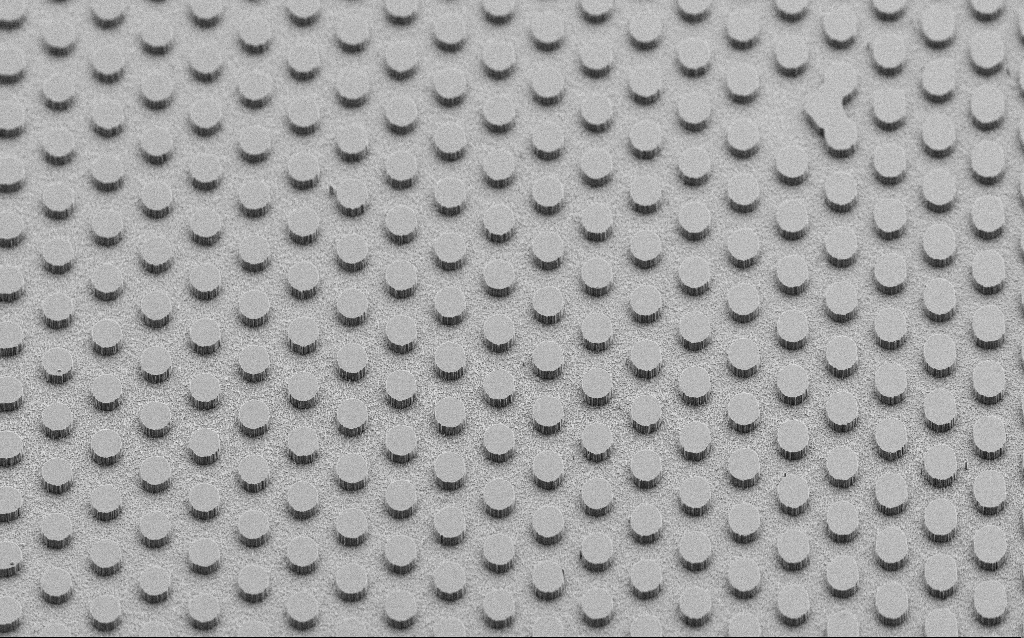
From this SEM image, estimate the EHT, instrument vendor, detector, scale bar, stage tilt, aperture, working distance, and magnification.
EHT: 5 kV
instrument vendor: Zeiss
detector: SE2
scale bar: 100000 nm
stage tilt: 45°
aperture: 30 µm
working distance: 7 mm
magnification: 0.6 K X